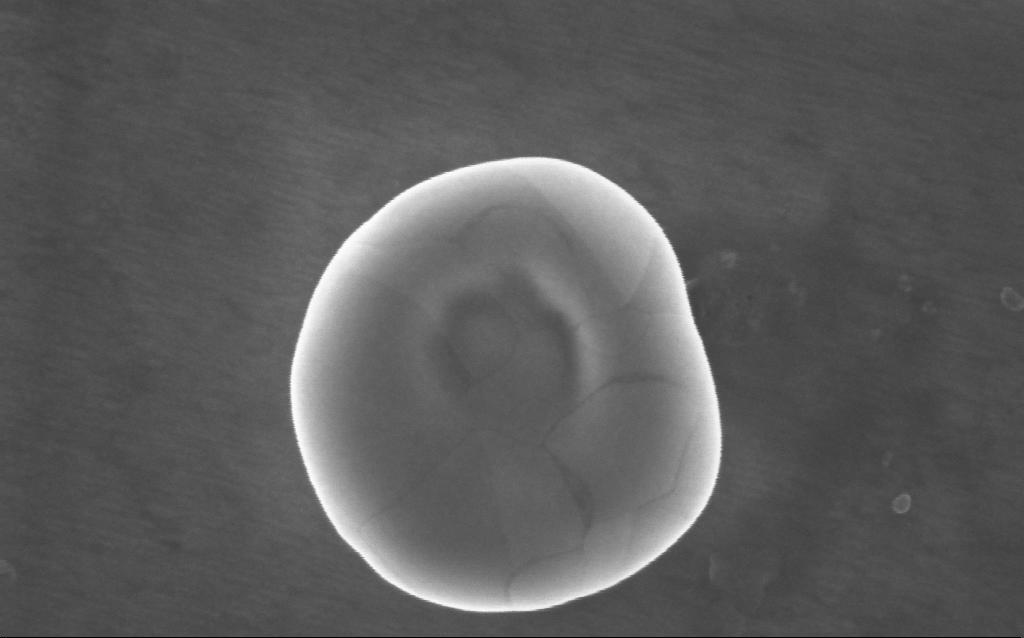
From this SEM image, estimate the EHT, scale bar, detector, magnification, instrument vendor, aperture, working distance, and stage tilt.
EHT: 5 kV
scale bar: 200 nm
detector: InLens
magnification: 232 K X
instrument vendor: Zeiss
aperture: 30 µm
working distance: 2 mm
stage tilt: -0°